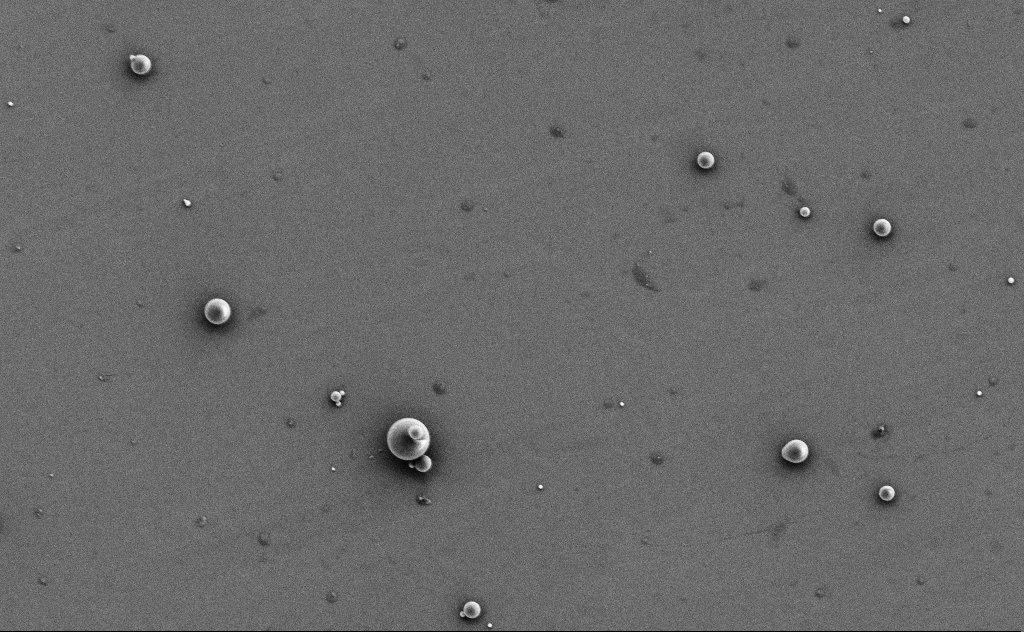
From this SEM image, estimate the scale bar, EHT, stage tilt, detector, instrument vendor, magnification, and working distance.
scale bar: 2000 nm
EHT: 3 kV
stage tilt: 0°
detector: SE2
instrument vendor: Zeiss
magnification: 7.47 K X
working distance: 11 mm